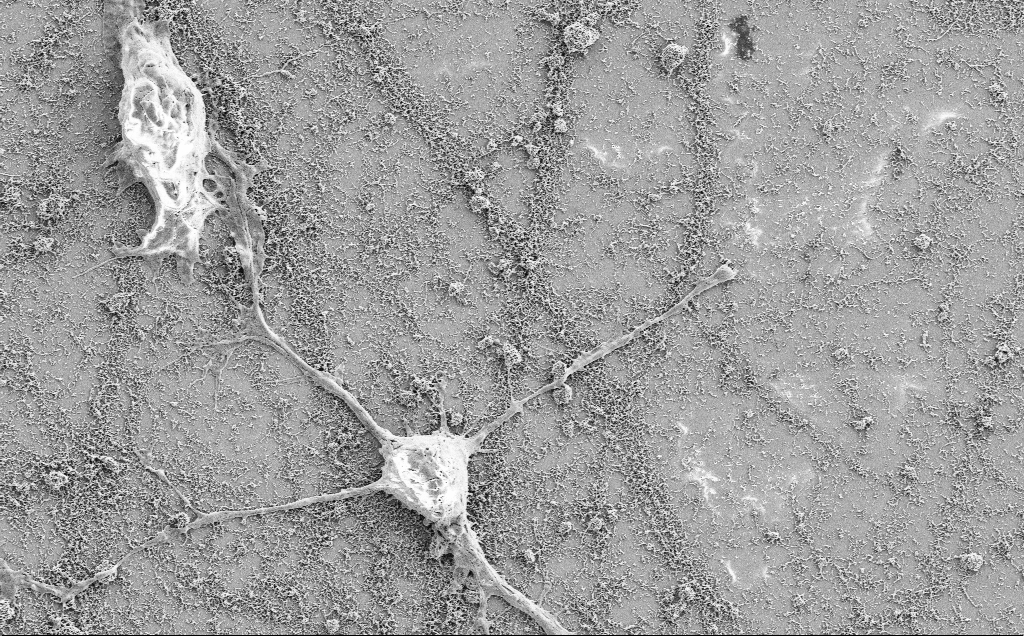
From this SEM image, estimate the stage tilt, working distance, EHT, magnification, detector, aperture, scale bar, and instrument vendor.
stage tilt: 0°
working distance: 7.1 mm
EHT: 2 kV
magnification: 5 K X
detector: SE2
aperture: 30 µm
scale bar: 10000 nm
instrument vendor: Zeiss